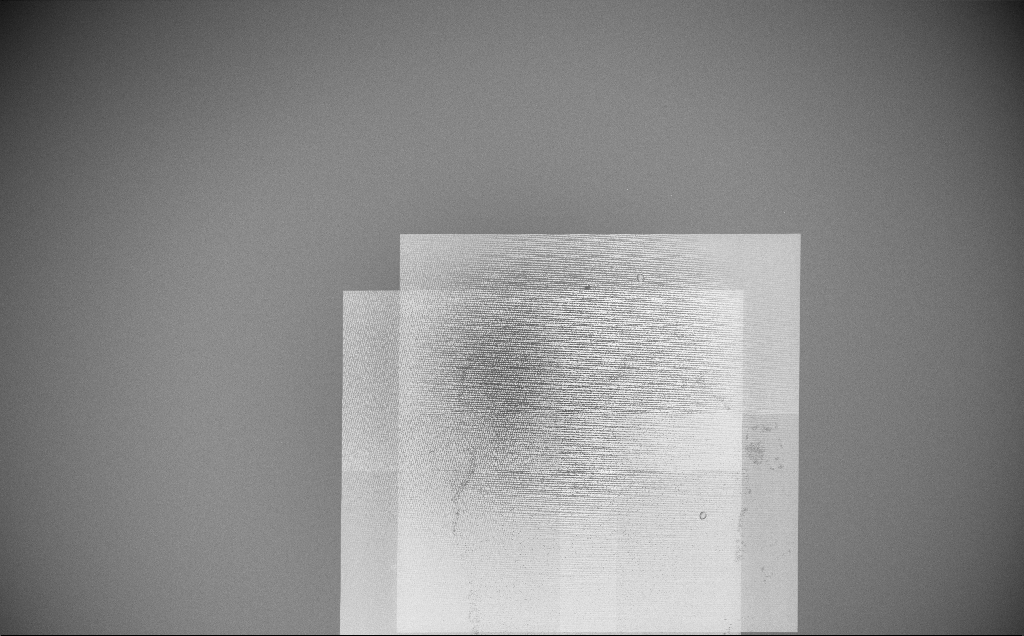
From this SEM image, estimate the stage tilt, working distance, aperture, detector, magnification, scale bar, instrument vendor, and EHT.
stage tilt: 0°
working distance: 6 mm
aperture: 30 µm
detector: InLens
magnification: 0.148 K X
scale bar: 100000 nm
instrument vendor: Zeiss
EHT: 10 kV